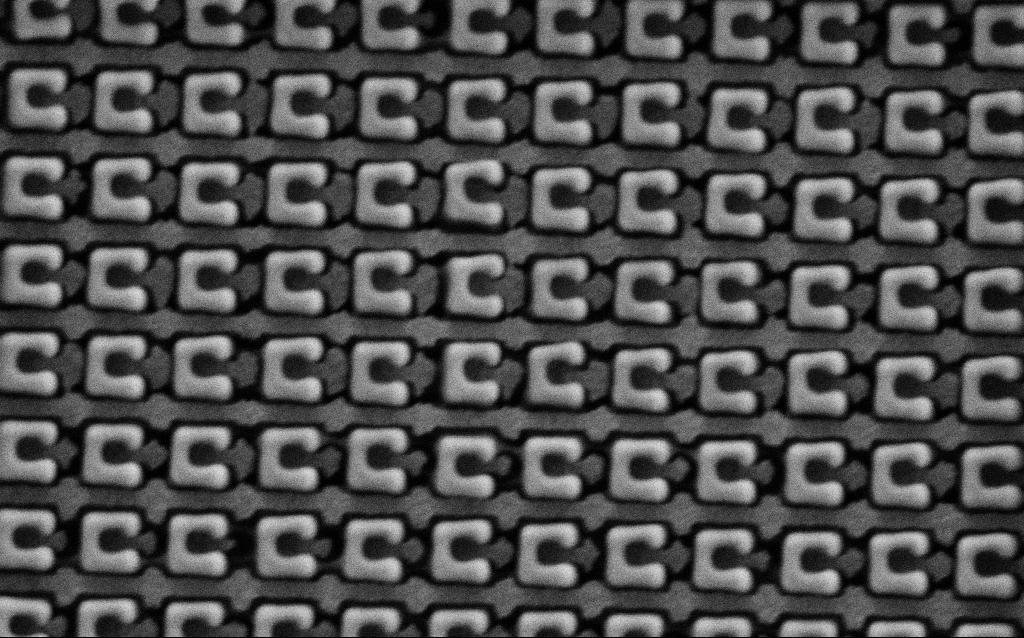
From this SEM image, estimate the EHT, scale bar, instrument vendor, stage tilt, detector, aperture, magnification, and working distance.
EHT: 1.5 kV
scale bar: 1000 nm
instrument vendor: Zeiss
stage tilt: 0°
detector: SE2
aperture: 30 µm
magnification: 69.73 K X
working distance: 4.9 mm